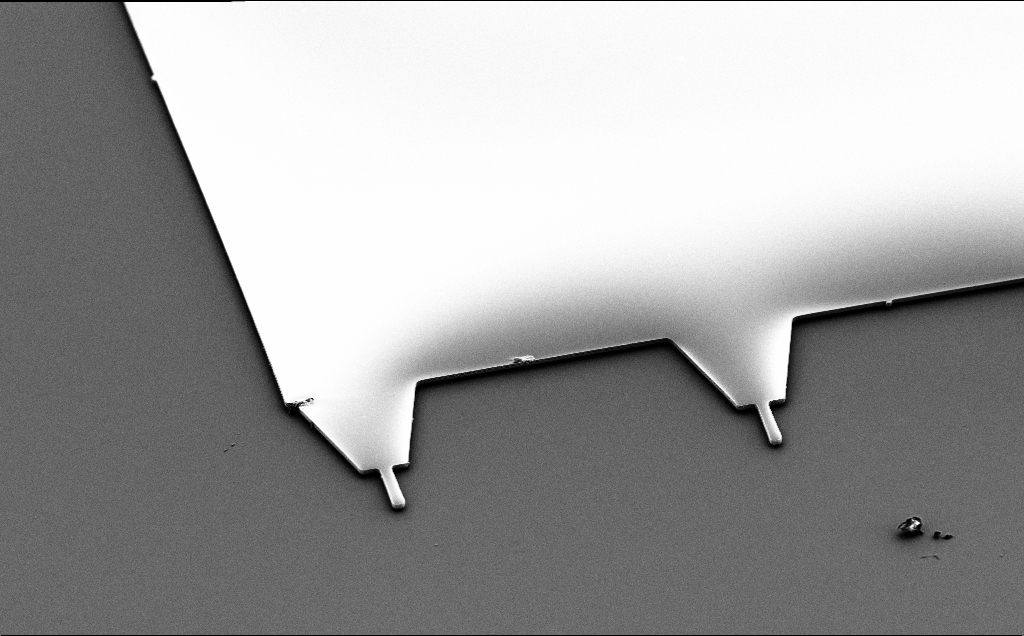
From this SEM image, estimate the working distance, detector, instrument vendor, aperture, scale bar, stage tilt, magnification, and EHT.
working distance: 10 mm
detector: SE2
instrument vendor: Zeiss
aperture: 30 µm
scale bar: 20000 nm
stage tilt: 50°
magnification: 0.895 K X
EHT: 5 kV